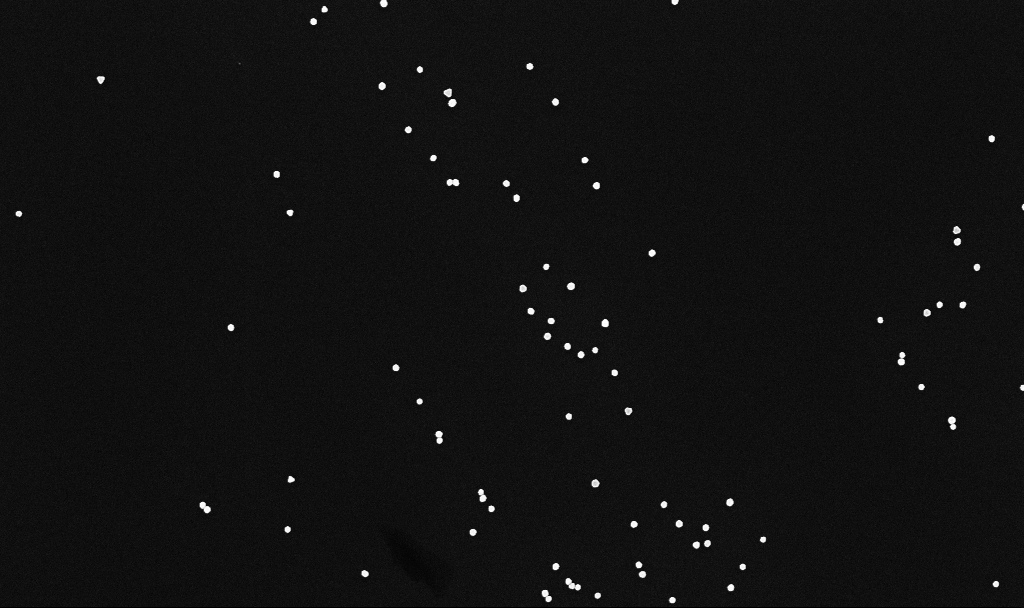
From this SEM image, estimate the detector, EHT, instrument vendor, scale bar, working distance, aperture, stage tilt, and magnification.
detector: InLens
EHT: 10 kV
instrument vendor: Zeiss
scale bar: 1000 nm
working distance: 3.4 mm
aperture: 30 µm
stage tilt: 0°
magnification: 38.82 K X